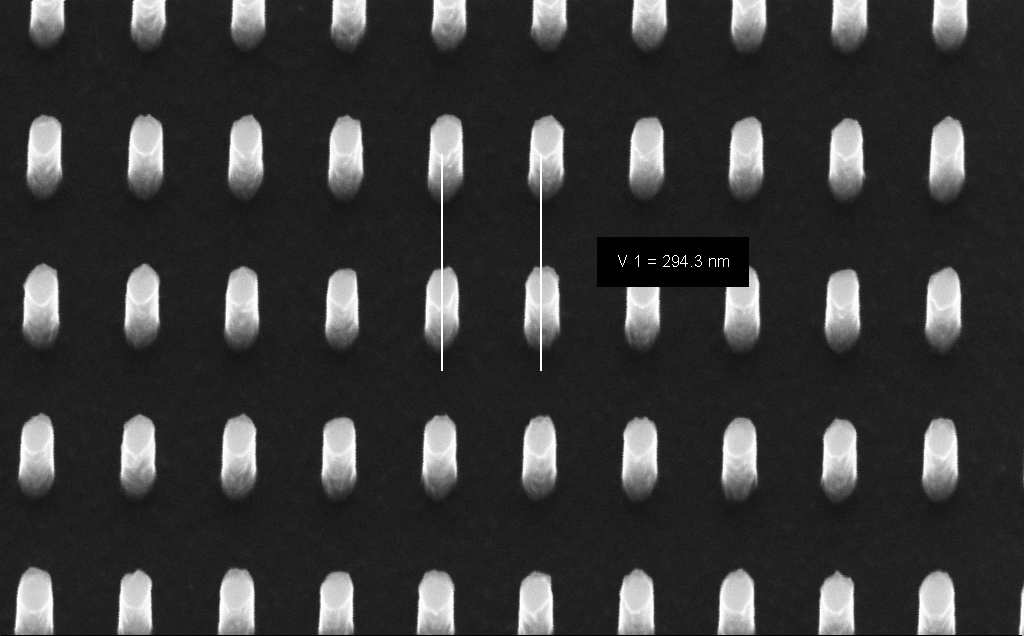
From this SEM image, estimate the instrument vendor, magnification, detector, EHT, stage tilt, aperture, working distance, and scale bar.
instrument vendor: Zeiss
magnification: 123.5 K X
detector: InLens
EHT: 10 kV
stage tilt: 30°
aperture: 30 µm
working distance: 5 mm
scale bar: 200 nm